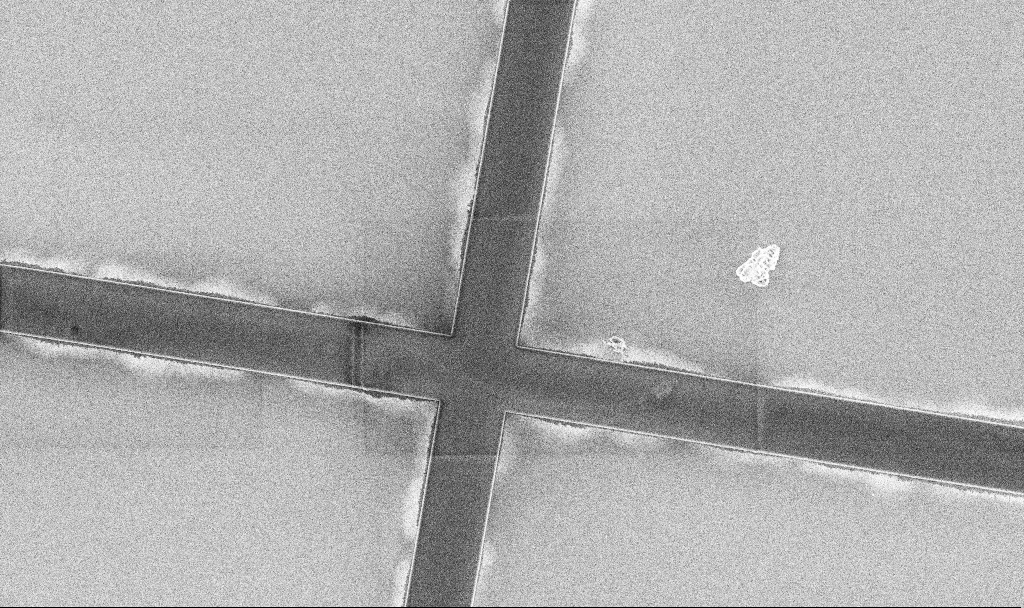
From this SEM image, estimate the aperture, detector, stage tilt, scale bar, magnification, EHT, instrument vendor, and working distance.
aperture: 30 µm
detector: InLens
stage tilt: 0°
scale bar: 20000 nm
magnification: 2.46 K X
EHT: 5 kV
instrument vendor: Zeiss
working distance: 5.8 mm